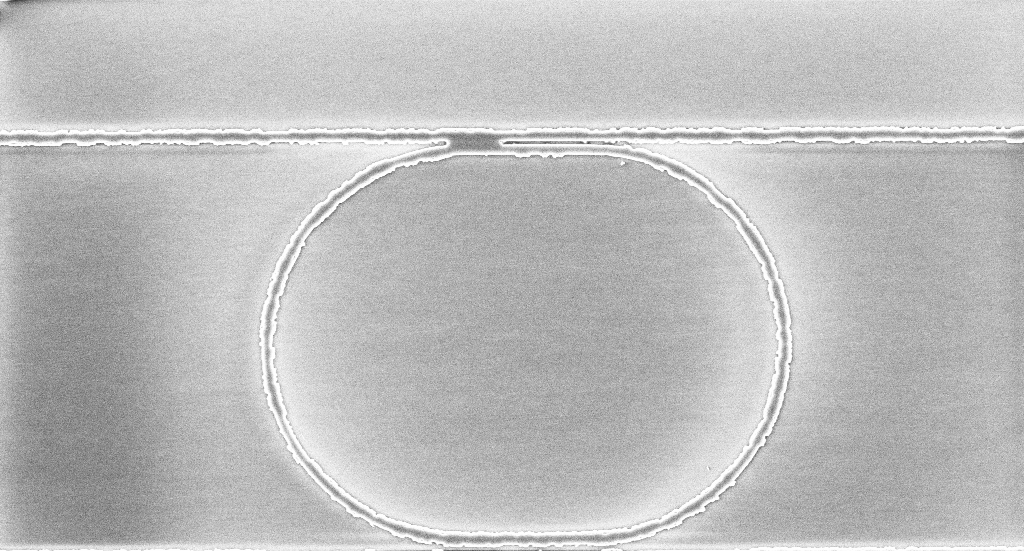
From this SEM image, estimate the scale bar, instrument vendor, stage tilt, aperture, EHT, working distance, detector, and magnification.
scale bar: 2000 nm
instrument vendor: Zeiss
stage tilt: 0°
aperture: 30 µm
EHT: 5 kV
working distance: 10.1 mm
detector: InLens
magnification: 7.49 K X